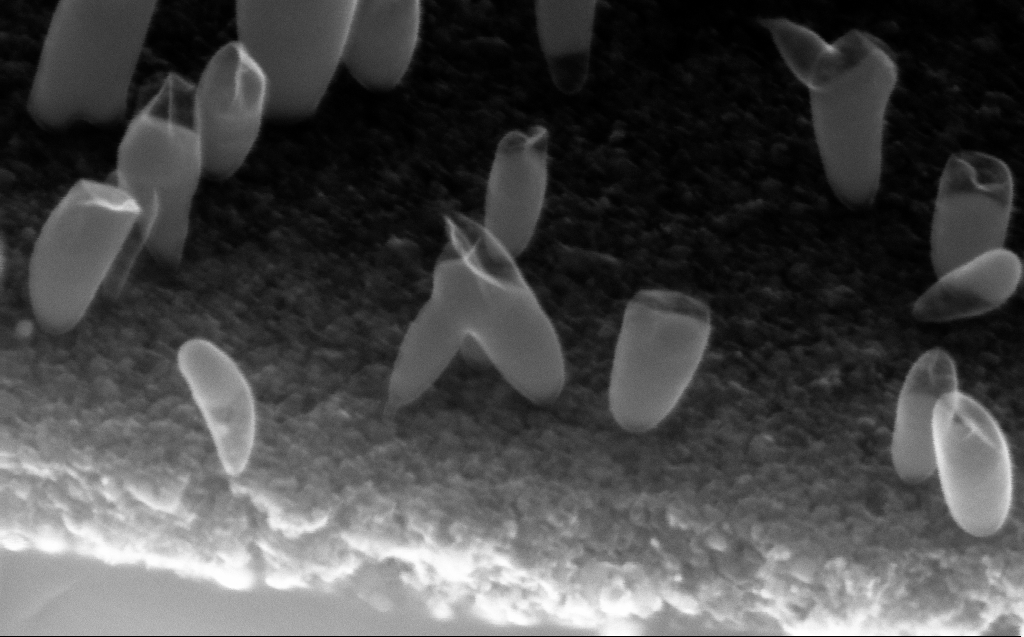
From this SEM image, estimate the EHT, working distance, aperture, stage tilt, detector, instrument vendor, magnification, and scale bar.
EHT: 10 kV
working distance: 5 mm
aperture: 30 µm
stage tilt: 45°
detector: InLens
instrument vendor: Zeiss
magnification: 252.27 K X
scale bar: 200 nm